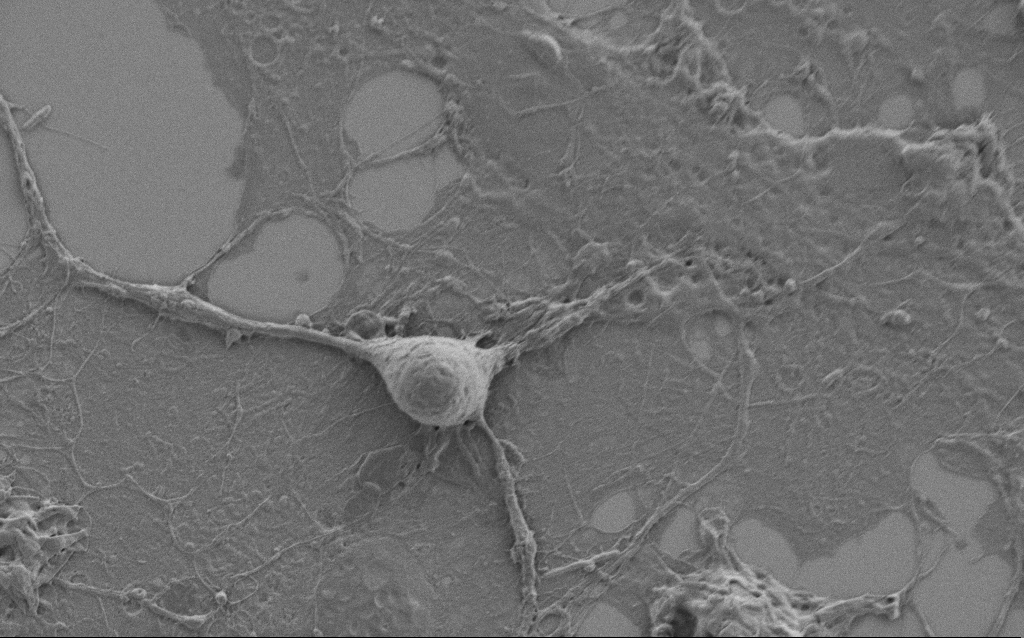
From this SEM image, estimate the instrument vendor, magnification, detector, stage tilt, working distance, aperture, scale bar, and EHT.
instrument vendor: Zeiss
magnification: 5 K X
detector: SE2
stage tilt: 0°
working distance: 5.9 mm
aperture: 30 µm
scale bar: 10000 nm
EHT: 1 kV